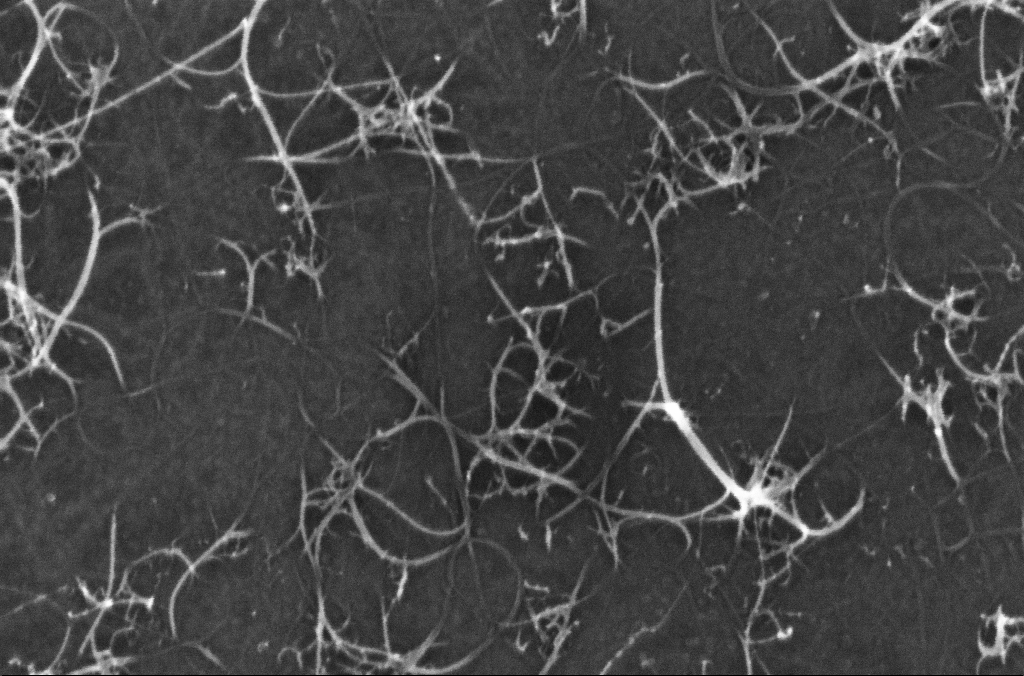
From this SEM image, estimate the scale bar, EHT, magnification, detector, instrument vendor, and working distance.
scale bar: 200 nm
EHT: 10 kV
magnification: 272.29 K X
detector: InLens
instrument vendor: Zeiss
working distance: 3.2 mm